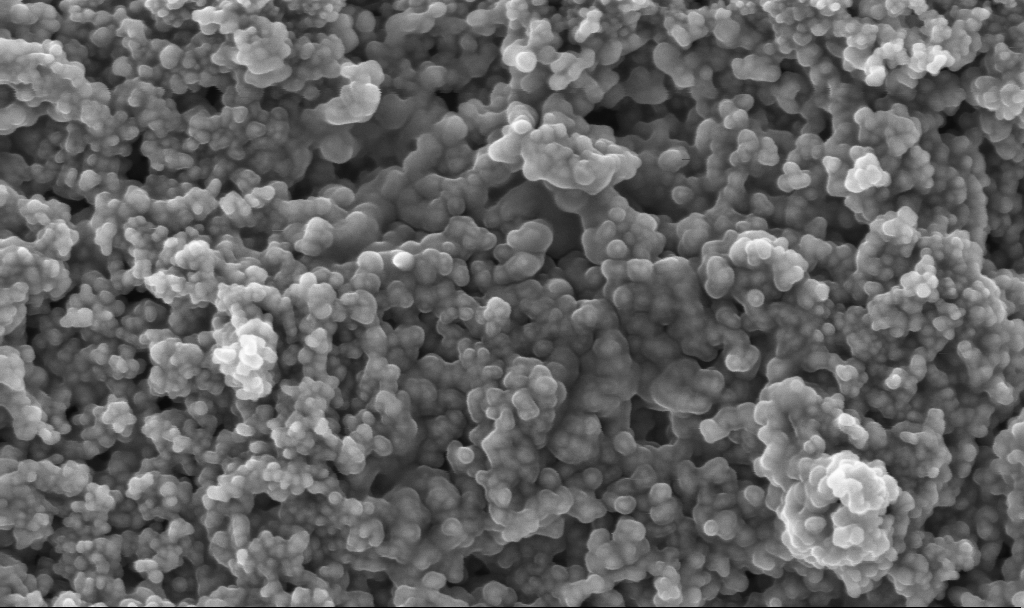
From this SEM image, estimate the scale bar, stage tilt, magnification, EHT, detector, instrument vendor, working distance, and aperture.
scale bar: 200 nm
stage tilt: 0°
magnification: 110.44 K X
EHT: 3 kV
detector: InLens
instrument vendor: Zeiss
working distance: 2.3 mm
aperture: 30 µm